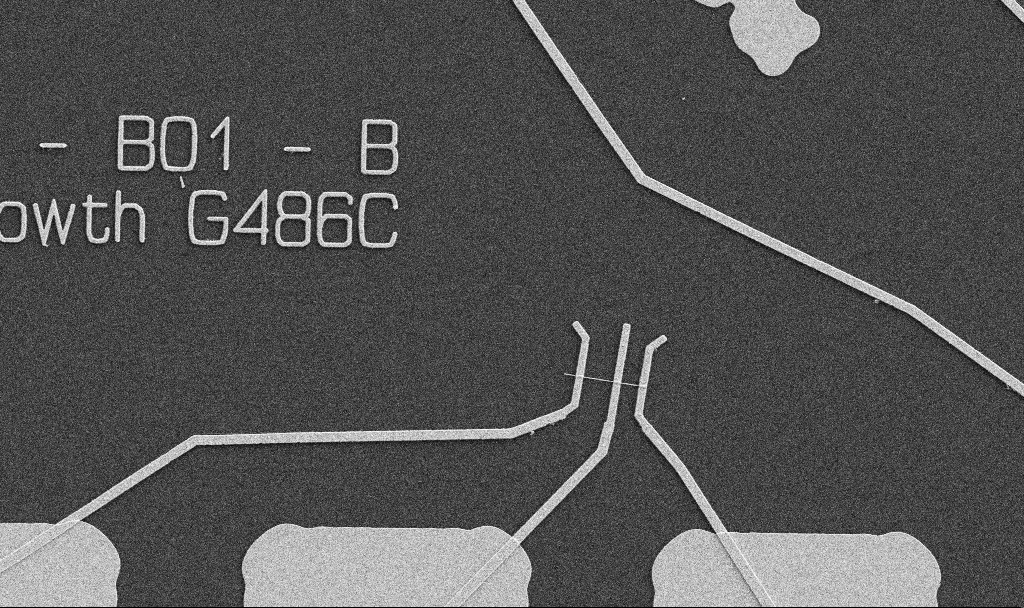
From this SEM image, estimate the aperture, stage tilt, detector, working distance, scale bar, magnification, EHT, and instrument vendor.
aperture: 30 µm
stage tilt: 0°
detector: SE2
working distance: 10.7 mm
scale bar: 10000 nm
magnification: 5 K X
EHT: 5 kV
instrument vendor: Zeiss